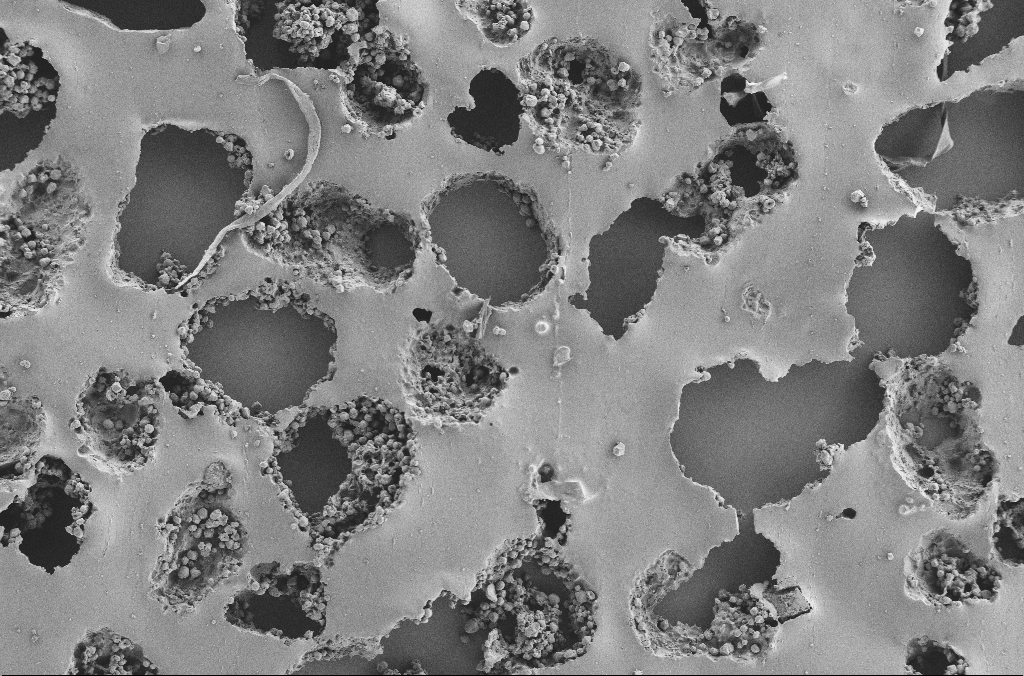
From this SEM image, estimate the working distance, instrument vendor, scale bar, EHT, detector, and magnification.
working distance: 3.7 mm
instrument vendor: Zeiss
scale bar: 100000 nm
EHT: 2 kV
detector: SE2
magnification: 0.25 K X